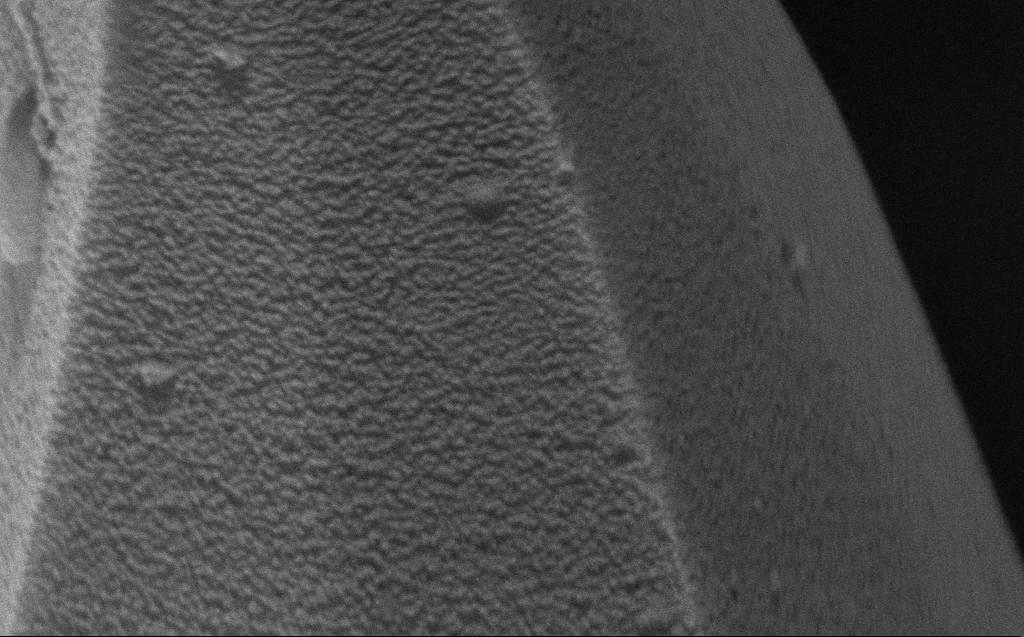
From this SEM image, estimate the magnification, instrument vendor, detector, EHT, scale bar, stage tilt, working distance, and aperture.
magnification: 134.45 K X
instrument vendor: Zeiss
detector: InLens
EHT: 4 kV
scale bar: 200 nm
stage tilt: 24.8°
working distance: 7 mm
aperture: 30 µm